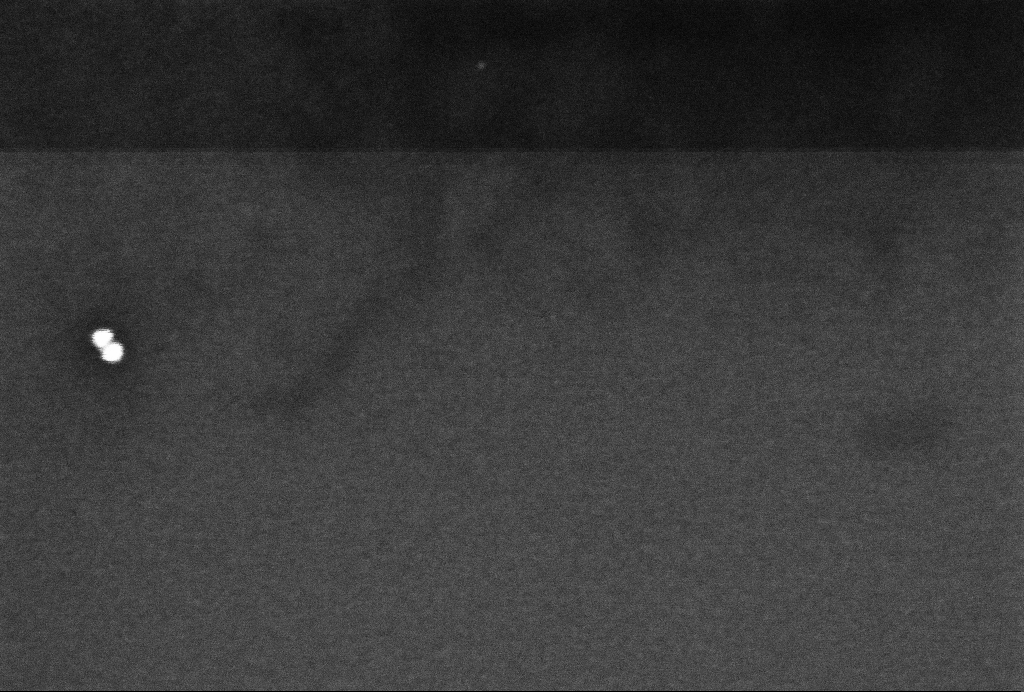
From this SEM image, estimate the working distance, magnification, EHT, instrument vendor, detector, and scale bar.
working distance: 3.3 mm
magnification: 283.27 K X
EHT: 2 kV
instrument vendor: Zeiss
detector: InLens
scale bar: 100 nm